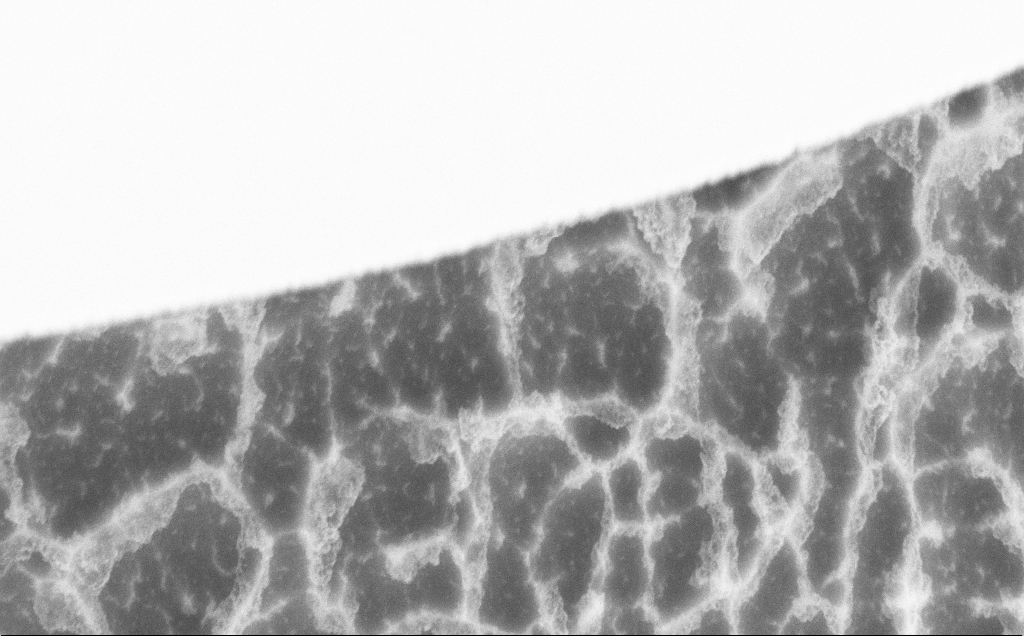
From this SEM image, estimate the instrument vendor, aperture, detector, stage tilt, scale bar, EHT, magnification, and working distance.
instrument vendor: Zeiss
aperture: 30 µm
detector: SE2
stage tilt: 45°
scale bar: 2000 nm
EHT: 5 kV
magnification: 33.68 K X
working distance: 8 mm